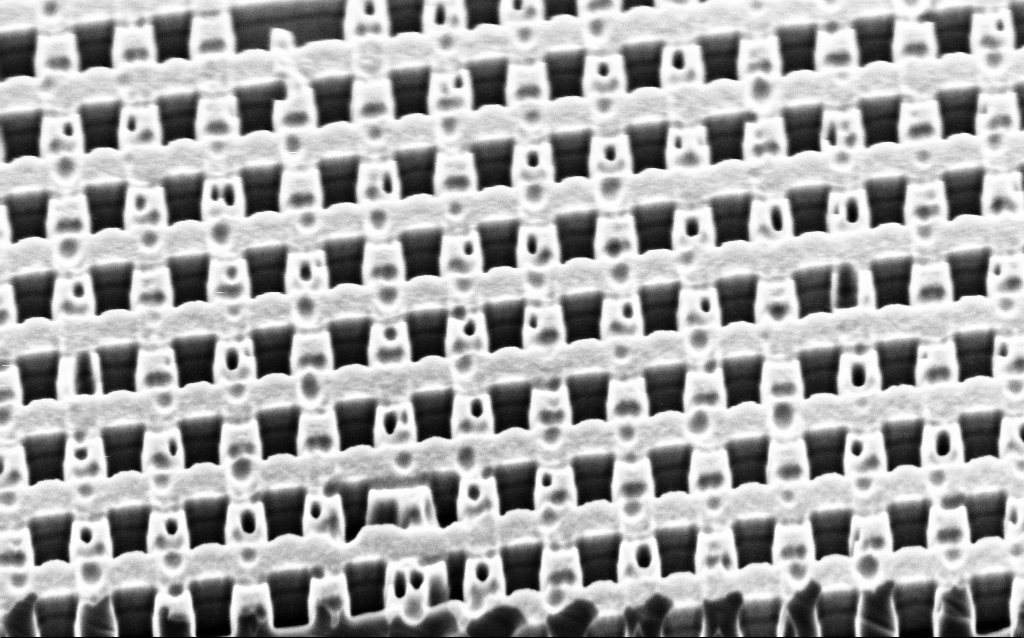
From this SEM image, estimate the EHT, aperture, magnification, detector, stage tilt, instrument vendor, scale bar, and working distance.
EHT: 2 kV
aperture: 30 µm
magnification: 57.33 K X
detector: InLens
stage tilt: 45°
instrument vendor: Zeiss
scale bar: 1000 nm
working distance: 3 mm